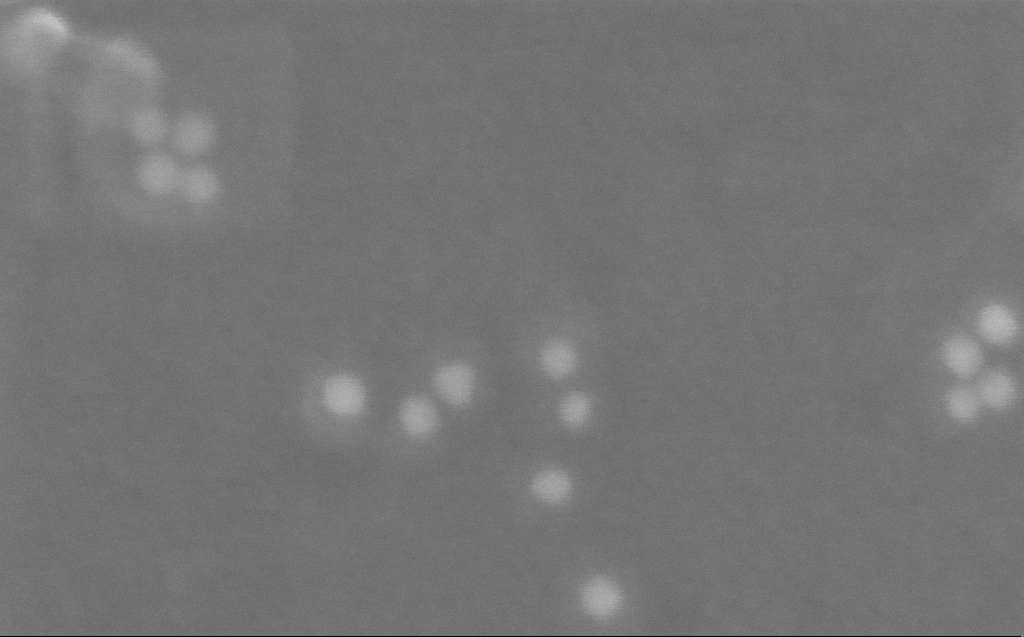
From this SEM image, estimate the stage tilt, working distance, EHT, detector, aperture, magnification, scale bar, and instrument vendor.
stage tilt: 0°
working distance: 3 mm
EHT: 10 kV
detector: InLens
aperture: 30 µm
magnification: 913.13 K X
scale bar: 20 nm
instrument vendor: Zeiss